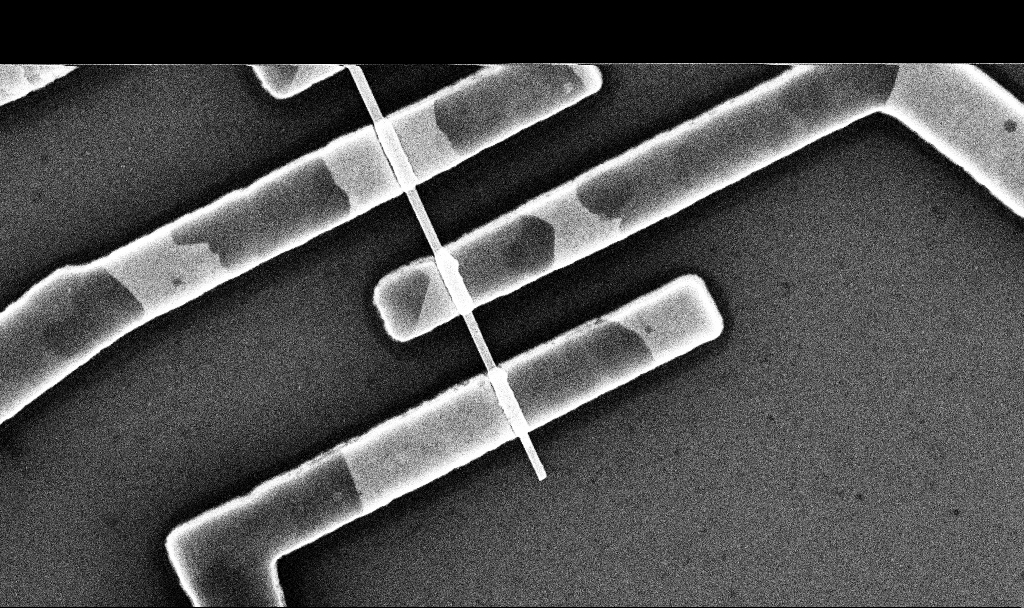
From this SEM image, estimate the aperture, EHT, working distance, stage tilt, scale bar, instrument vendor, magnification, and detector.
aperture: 30 µm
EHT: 10 kV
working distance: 6.8 mm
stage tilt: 0°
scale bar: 1000 nm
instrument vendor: Zeiss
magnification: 43.28 K X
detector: InLens